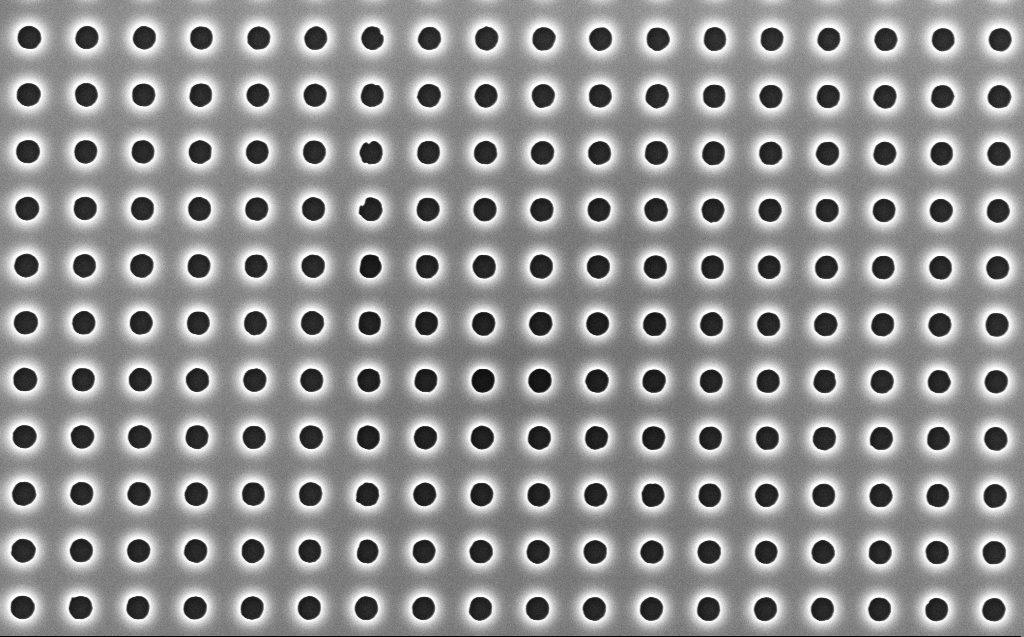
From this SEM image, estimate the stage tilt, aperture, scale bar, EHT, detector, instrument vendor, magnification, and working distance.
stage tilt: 0°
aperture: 30 µm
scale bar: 2000 nm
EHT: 5 kV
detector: InLens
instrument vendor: Zeiss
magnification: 20 K X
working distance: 7 mm